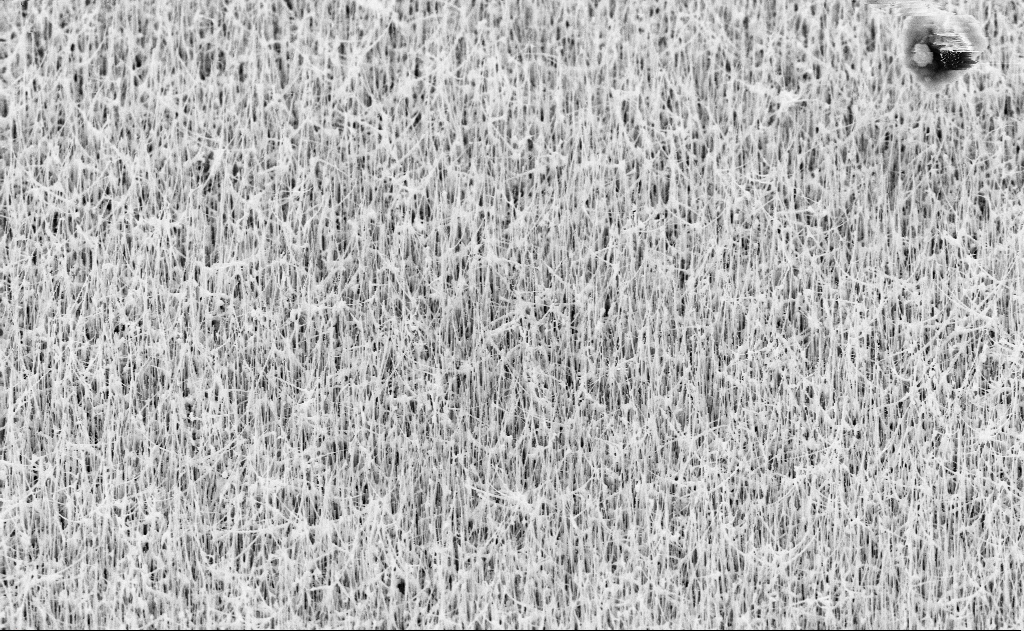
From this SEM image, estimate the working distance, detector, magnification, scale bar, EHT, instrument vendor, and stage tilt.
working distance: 14 mm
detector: SE2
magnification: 10 K X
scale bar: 2000 nm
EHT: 10 kV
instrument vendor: Zeiss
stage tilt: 45°